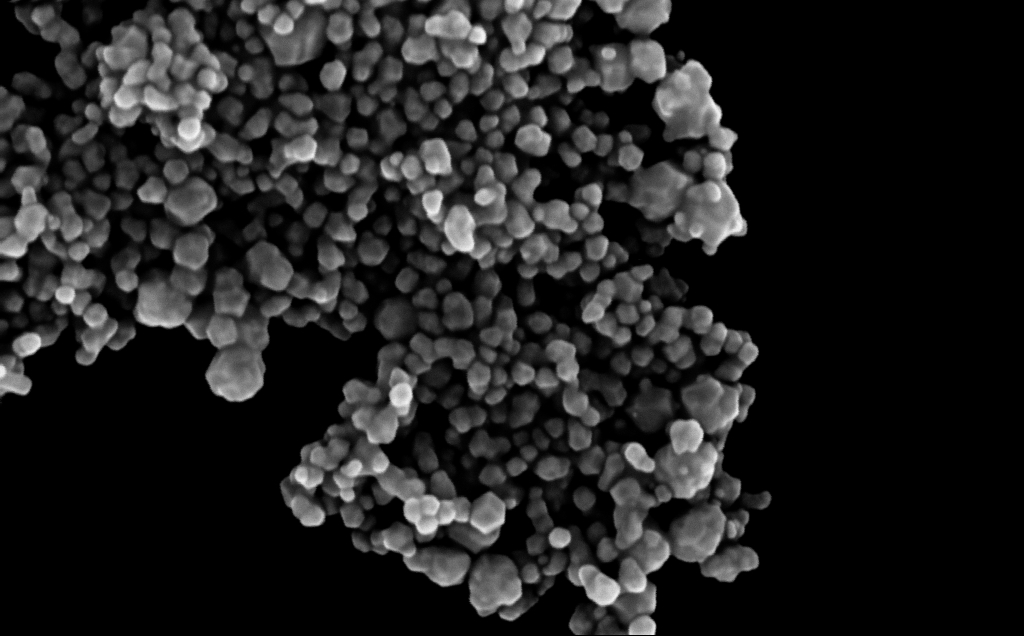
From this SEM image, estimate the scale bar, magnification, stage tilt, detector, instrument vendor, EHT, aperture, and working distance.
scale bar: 200 nm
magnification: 222.8 K X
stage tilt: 0°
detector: InLens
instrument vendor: Zeiss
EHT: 10 kV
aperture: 30 µm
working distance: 3 mm